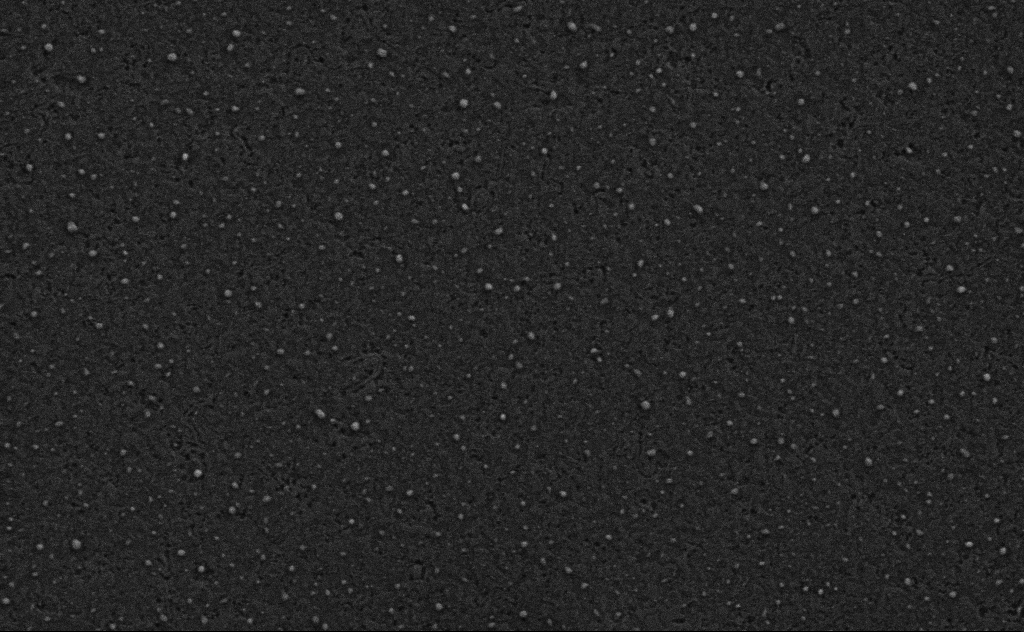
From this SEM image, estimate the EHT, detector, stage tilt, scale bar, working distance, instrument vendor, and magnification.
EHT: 3 kV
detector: SE2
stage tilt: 0°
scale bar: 1000 nm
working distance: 4 mm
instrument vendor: Zeiss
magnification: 62.19 K X